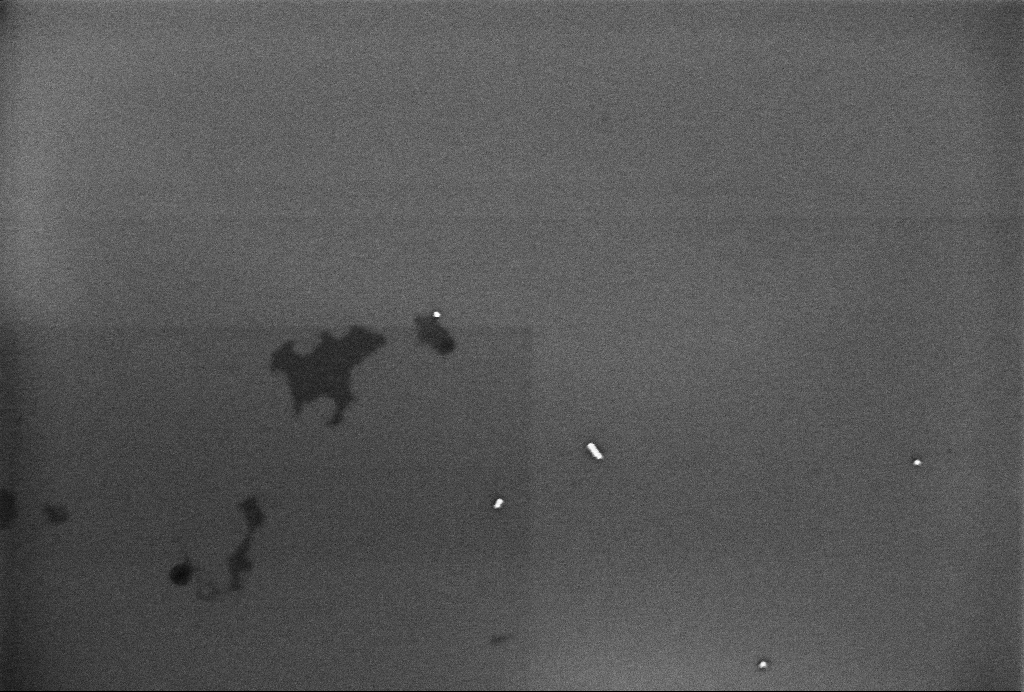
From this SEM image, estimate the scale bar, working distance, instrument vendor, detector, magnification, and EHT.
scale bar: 200 nm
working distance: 3.3 mm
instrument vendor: Zeiss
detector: InLens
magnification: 71 K X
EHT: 1 kV